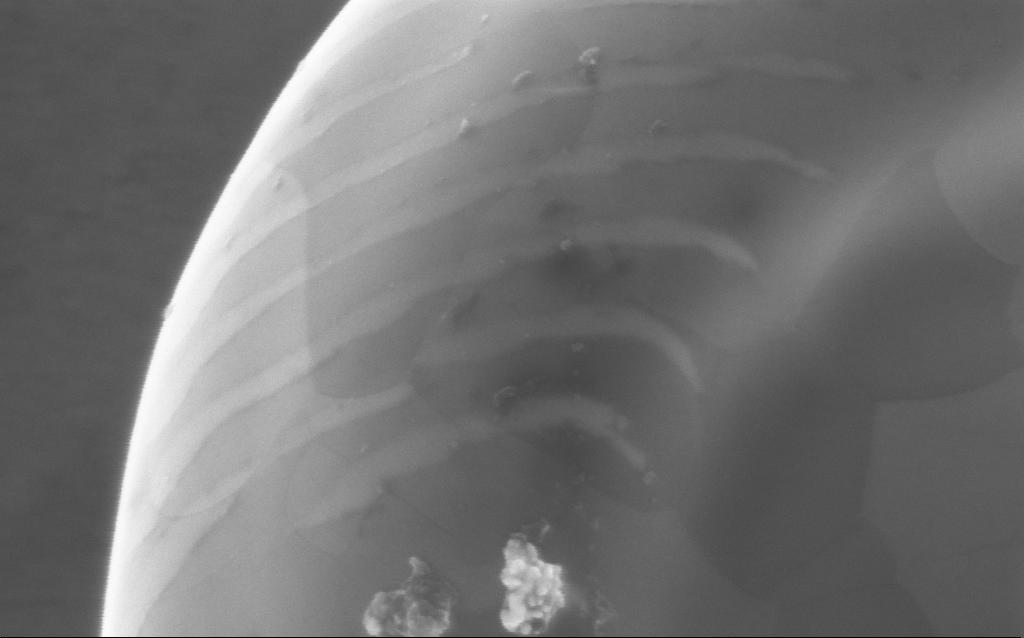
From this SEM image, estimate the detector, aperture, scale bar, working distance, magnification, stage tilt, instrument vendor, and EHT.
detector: InLens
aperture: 30 µm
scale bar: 200 nm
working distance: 4 mm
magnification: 203.5 K X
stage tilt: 0°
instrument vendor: Zeiss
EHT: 5 kV